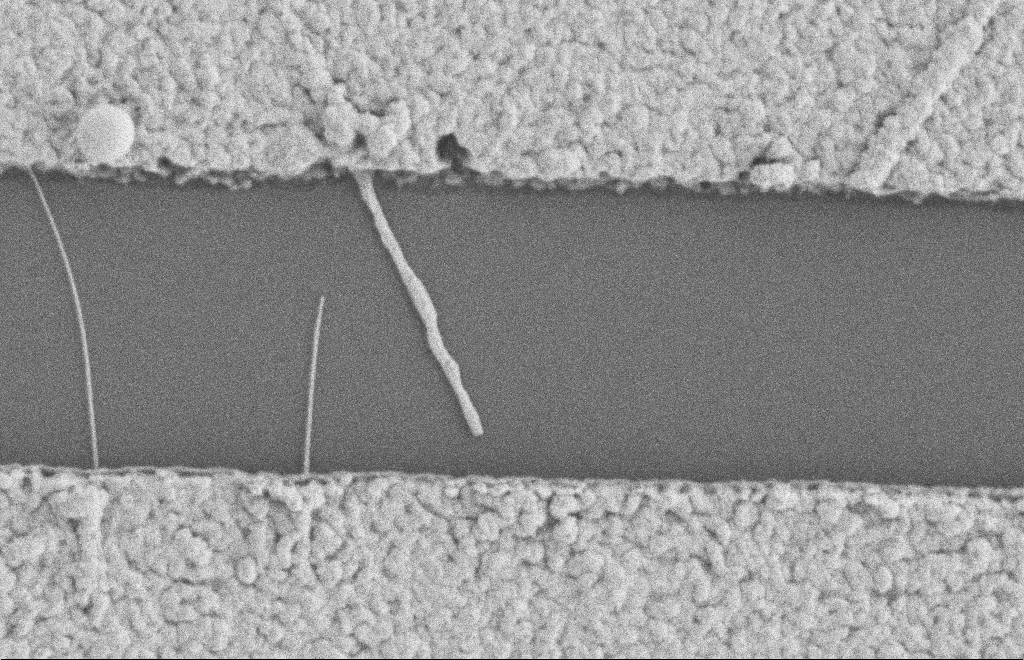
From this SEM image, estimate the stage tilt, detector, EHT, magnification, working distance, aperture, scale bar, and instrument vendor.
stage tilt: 0°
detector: SE2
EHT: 2 kV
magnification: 45.07 K X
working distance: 8 mm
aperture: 20 µm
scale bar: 1000 nm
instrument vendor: Zeiss